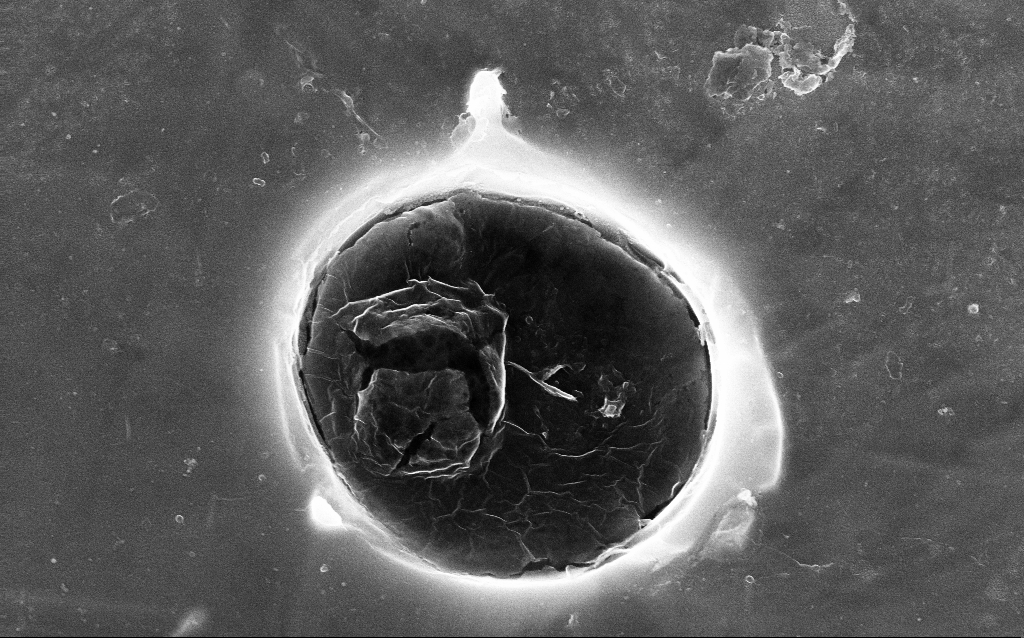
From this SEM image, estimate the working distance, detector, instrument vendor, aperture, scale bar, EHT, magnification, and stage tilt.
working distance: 5.7 mm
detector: InLens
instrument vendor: Zeiss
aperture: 30 µm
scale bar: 2000 nm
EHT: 20 kV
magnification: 28.62 K X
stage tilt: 21.2°